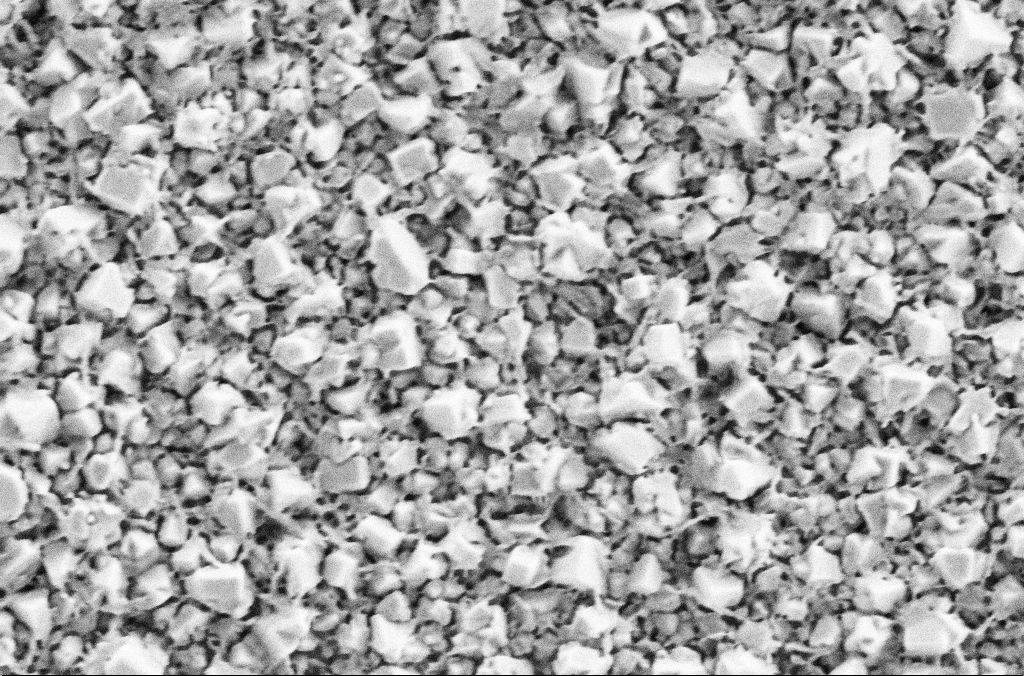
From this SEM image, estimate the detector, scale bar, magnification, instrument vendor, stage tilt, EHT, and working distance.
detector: SE2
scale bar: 1000 nm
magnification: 50 K X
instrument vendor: Zeiss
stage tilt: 0°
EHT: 2 kV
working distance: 1.9 mm